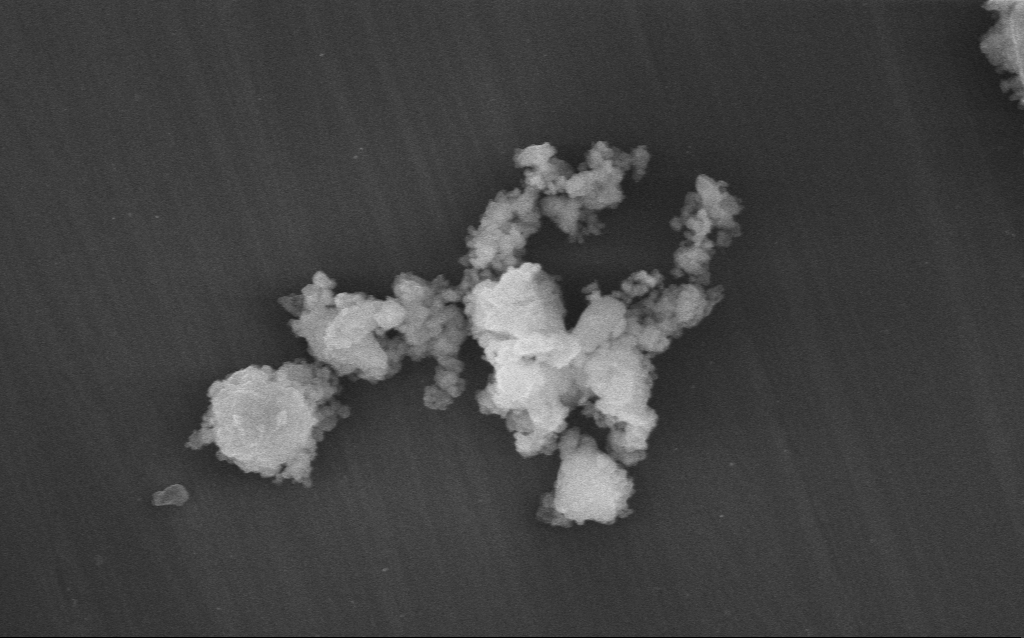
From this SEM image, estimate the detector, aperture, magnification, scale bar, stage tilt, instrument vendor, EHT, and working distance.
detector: InLens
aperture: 30 µm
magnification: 27.66 K X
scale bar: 2000 nm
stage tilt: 0°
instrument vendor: Zeiss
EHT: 10 kV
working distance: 4 mm